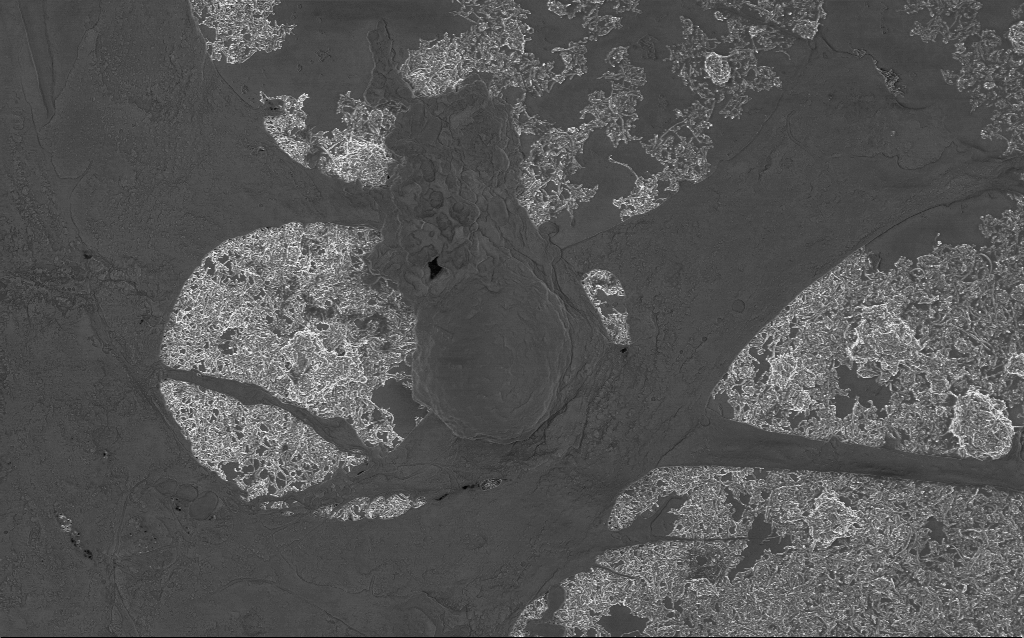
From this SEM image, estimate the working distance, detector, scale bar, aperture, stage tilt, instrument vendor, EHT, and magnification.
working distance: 6 mm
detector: InLens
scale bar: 2000 nm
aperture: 30 µm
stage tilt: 0°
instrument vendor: Zeiss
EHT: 1 kV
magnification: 10 K X